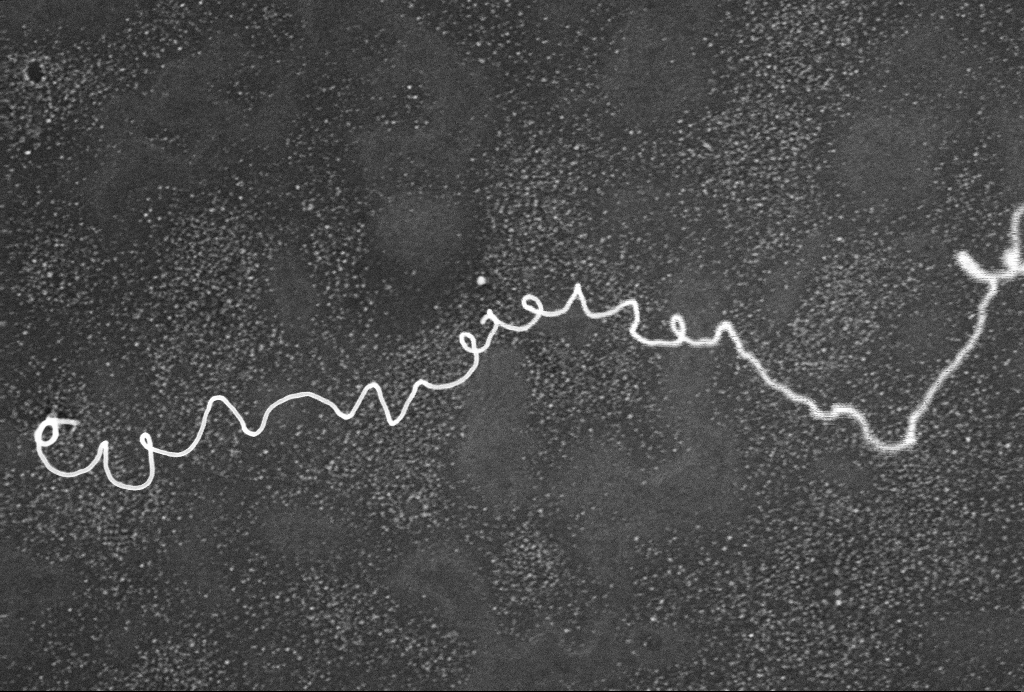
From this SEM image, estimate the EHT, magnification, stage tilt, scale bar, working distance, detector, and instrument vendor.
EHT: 10 kV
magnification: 38.58 K X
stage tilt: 0°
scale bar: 1000 nm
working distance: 3.1 mm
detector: InLens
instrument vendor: Zeiss